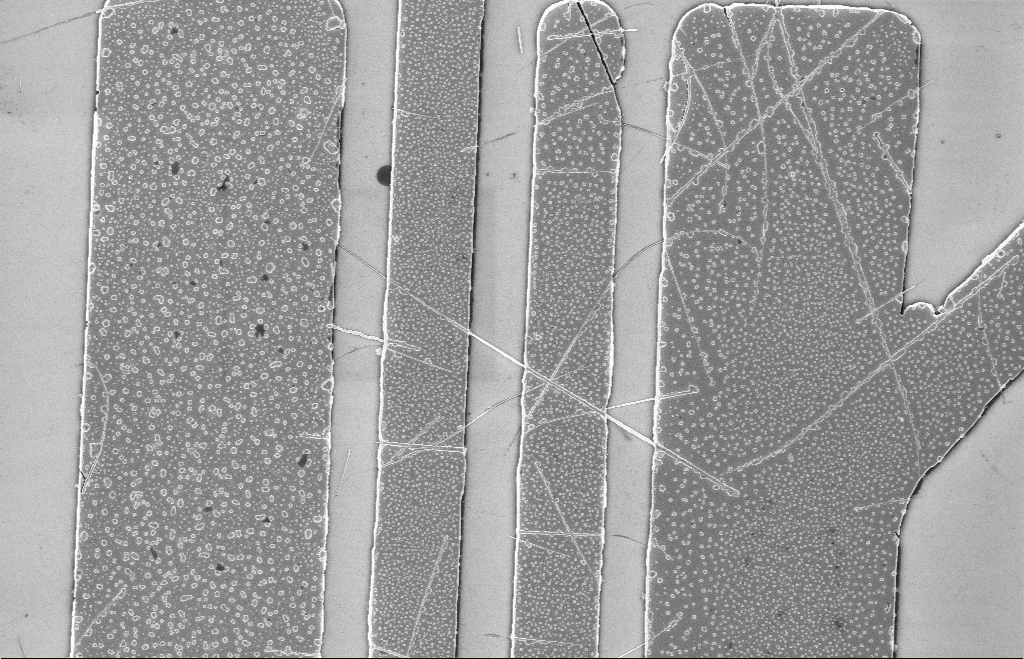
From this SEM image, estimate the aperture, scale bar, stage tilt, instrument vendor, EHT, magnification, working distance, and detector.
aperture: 20 µm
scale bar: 2000 nm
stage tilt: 0°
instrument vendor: Zeiss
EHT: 5 kV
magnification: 8.48 K X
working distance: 8 mm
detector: InLens